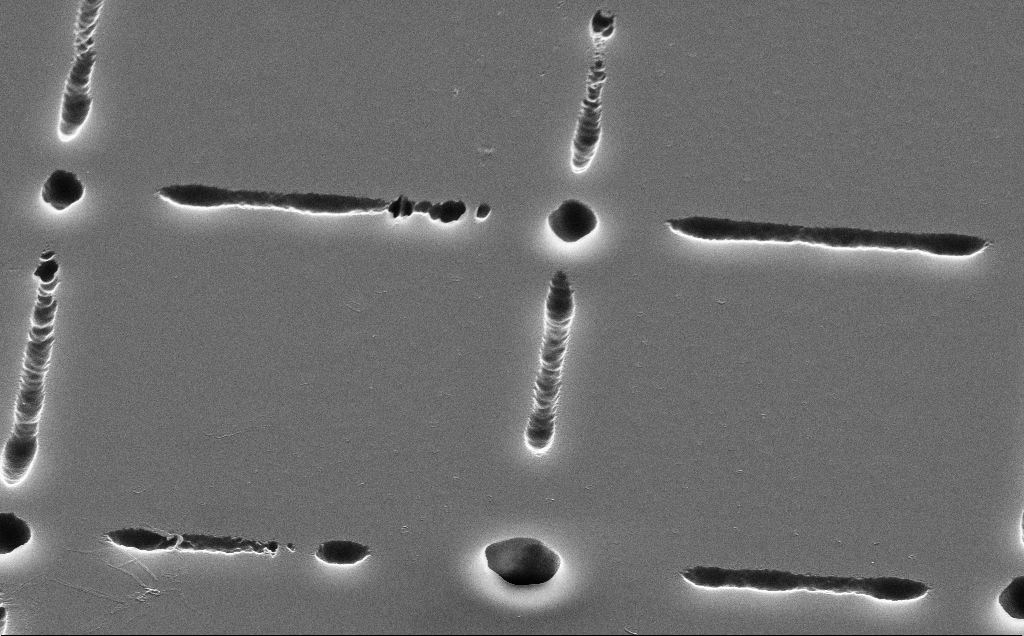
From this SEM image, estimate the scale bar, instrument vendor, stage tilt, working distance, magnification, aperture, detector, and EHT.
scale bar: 2000 nm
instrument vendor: Zeiss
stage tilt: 45°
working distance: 15 mm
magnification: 9.12 K X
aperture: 30 µm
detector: SE2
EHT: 10 kV